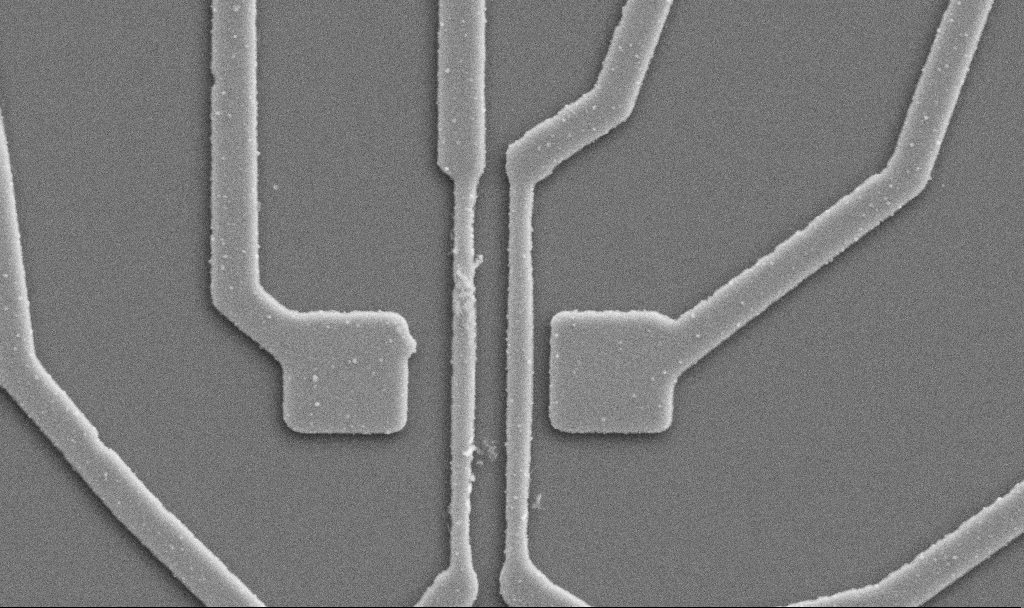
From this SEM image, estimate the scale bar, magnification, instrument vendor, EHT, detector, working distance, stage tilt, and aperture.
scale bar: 2000 nm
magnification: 20 K X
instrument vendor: Zeiss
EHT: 5 kV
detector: SE2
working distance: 10.7 mm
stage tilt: -0°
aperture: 30 µm